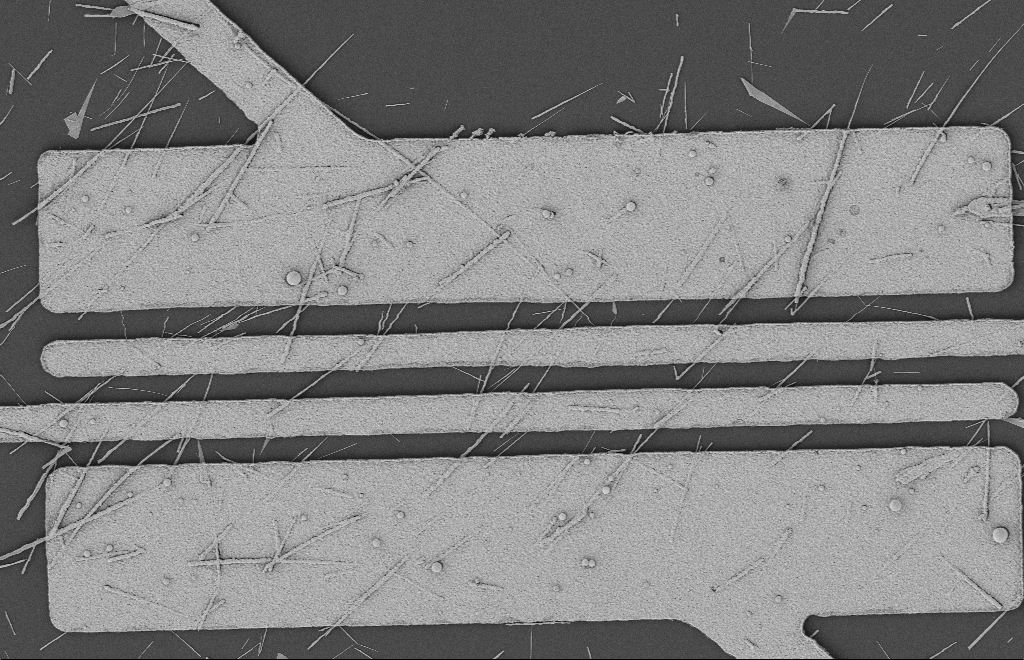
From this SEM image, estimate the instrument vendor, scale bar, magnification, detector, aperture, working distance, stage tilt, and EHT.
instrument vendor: Zeiss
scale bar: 2000 nm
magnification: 5.83 K X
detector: SE2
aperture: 20 µm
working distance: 12 mm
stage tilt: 0°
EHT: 2 kV